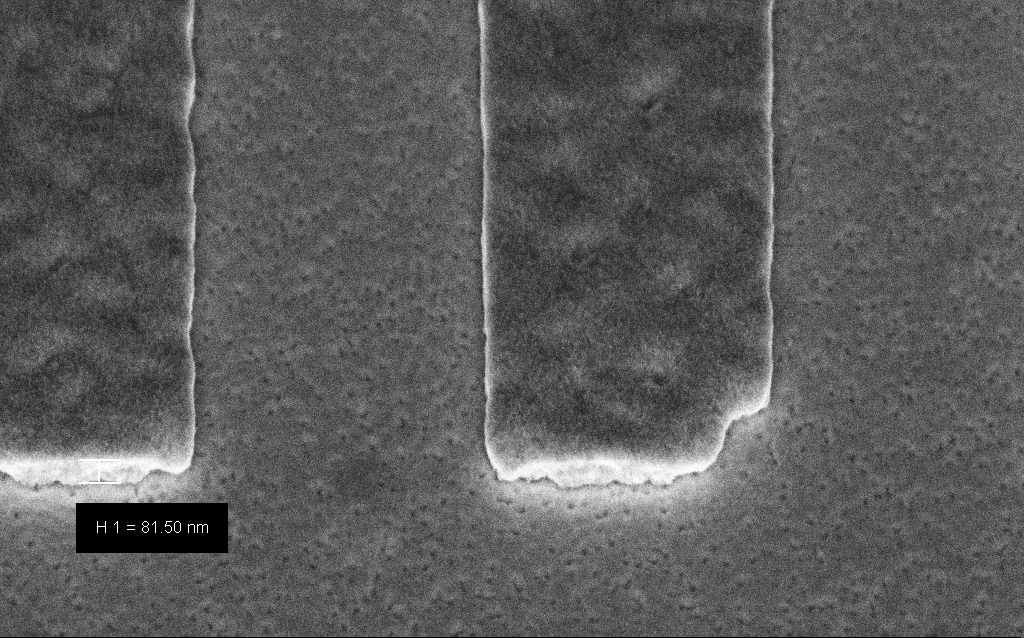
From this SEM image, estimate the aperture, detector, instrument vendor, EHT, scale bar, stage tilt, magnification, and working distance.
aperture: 30 µm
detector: InLens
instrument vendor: Zeiss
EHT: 3 kV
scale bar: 200 nm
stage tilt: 45°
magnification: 103.63 K X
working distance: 6.6 mm